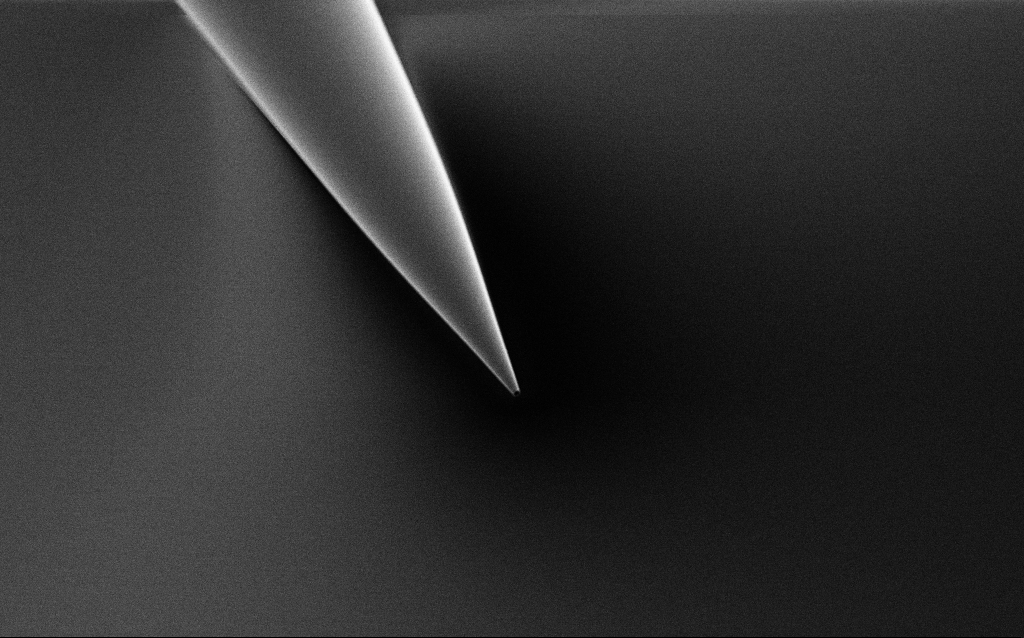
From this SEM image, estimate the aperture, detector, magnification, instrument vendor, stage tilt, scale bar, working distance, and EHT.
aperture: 30 µm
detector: SE2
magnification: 1 K X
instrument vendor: Zeiss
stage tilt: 45°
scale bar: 20000 nm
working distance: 7 mm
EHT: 1 kV